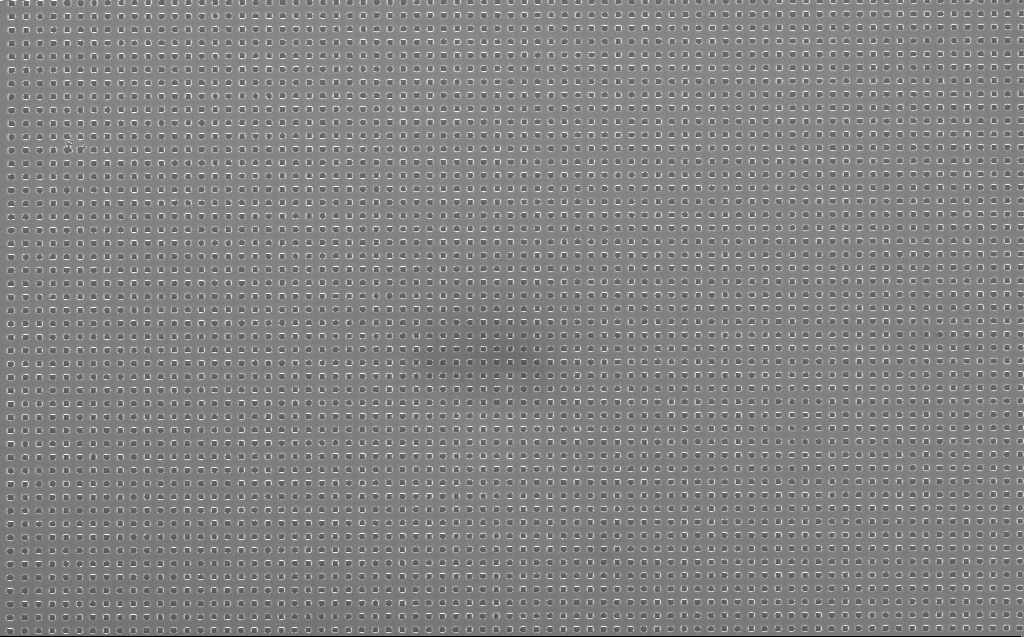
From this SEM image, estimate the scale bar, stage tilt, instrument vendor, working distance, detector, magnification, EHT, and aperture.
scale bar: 2000 nm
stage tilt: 0°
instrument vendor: Zeiss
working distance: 5 mm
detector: InLens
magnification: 10 K X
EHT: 10 kV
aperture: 30 µm